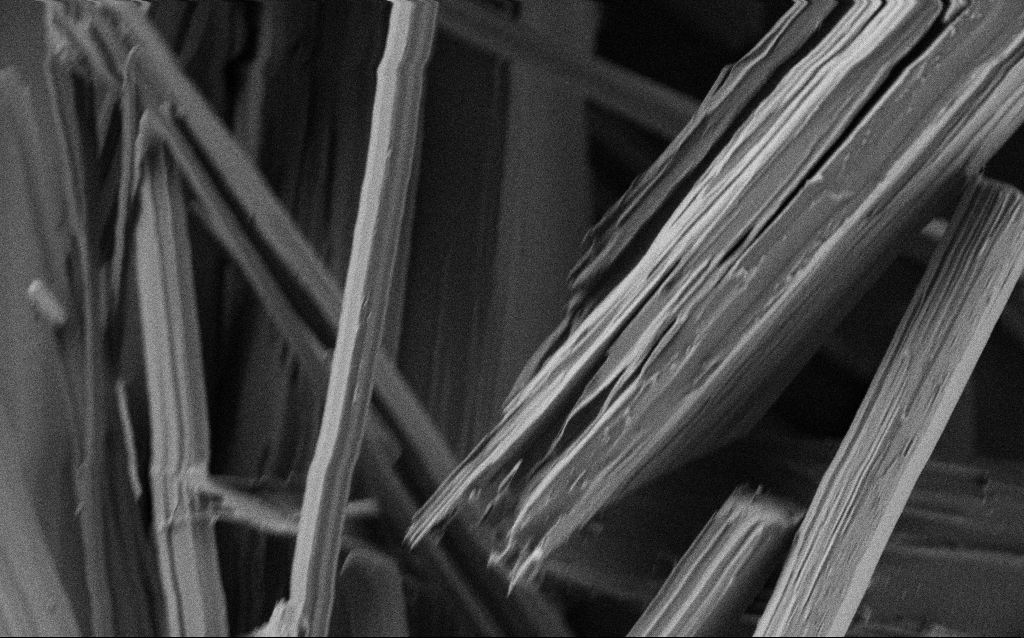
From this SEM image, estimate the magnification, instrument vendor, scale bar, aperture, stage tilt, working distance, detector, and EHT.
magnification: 15 K X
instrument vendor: Zeiss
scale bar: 1000 nm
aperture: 30 µm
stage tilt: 0°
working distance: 4.2 mm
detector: SE2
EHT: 0.9 kV